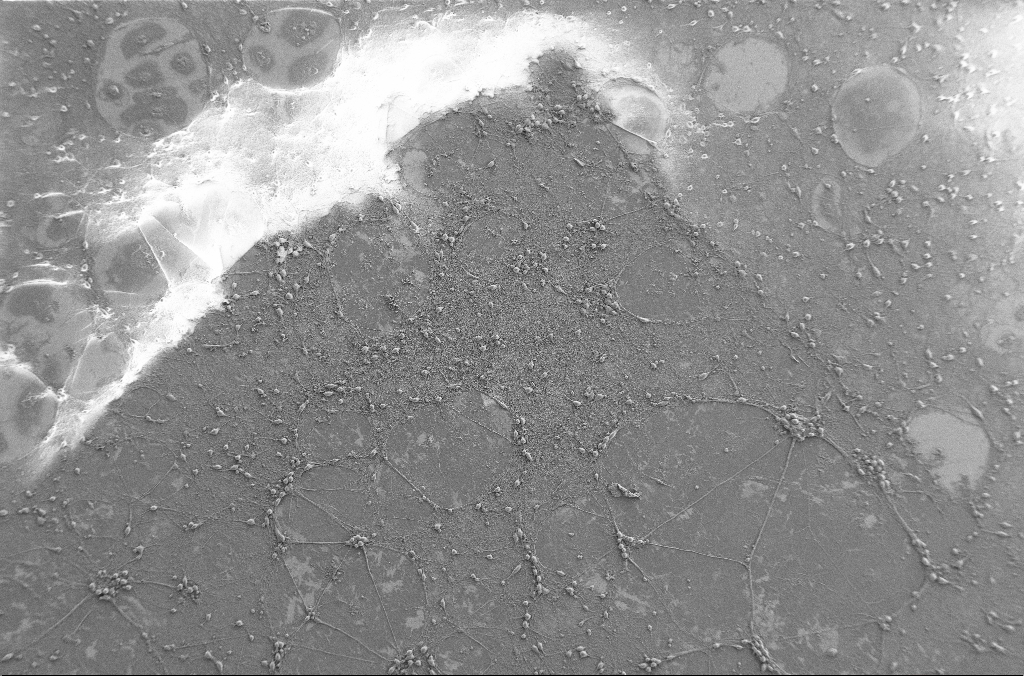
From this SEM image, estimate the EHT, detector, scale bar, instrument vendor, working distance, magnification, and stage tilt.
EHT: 2 kV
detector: SE2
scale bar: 100000 nm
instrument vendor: Zeiss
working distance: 4.1 mm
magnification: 0.25 K X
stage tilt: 0°